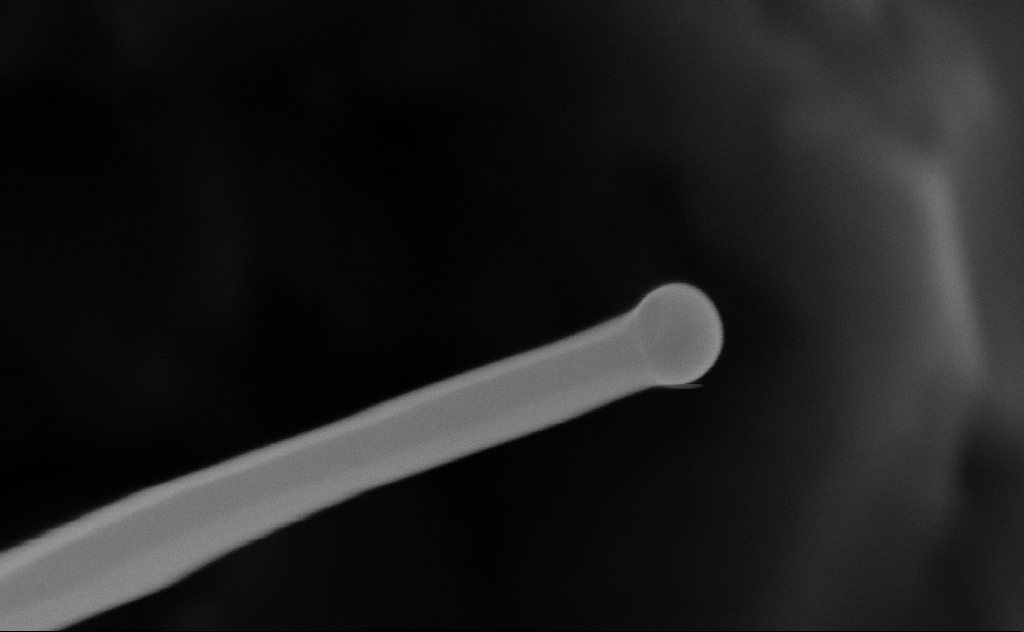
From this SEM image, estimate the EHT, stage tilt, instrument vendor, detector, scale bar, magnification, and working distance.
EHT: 10 kV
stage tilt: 0°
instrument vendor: Zeiss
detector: InLens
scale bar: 200 nm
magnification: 283.44 K X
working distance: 6 mm